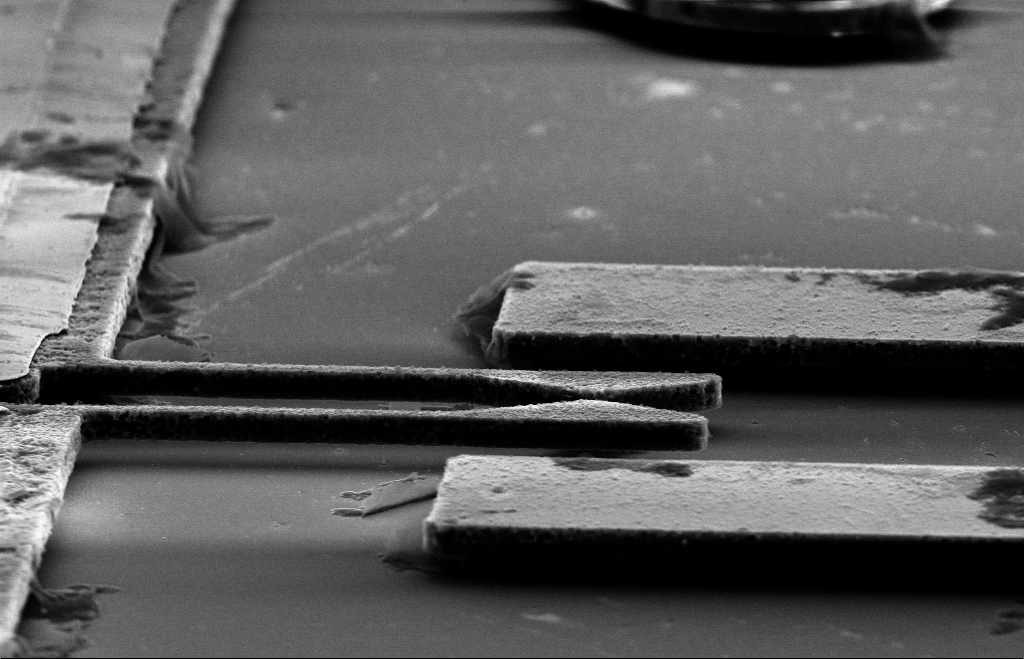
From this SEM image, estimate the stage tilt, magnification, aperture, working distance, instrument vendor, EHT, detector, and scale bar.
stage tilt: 70°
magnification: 4.57 K X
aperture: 30 µm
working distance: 11 mm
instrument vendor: Zeiss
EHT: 10 kV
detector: SE2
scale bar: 10000 nm